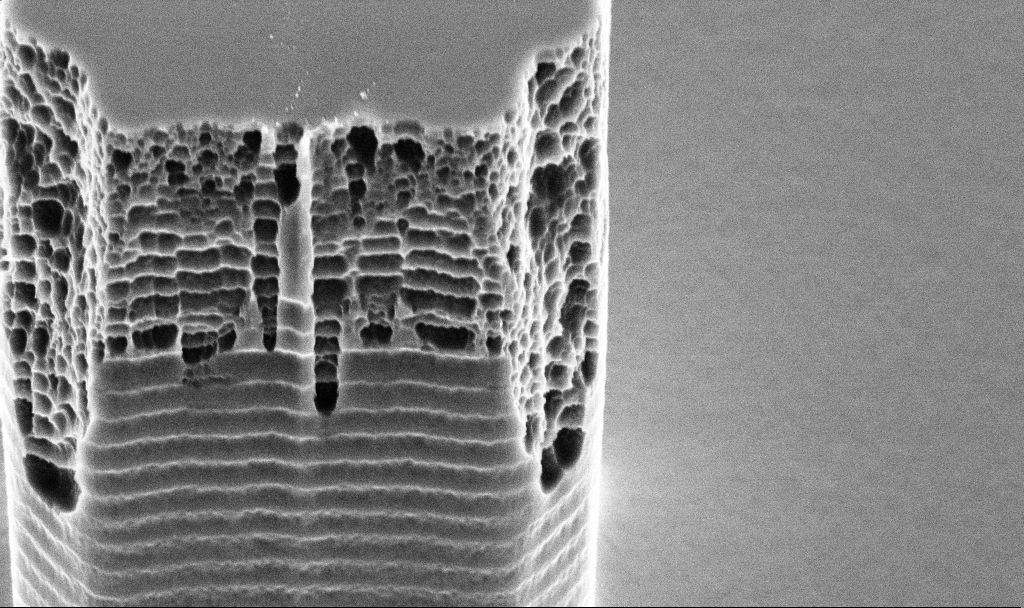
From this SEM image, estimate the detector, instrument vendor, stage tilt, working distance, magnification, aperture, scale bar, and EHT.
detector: SE2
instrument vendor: Zeiss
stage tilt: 45°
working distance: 11.2 mm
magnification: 22.53 K X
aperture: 30 µm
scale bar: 2000 nm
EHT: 5 kV